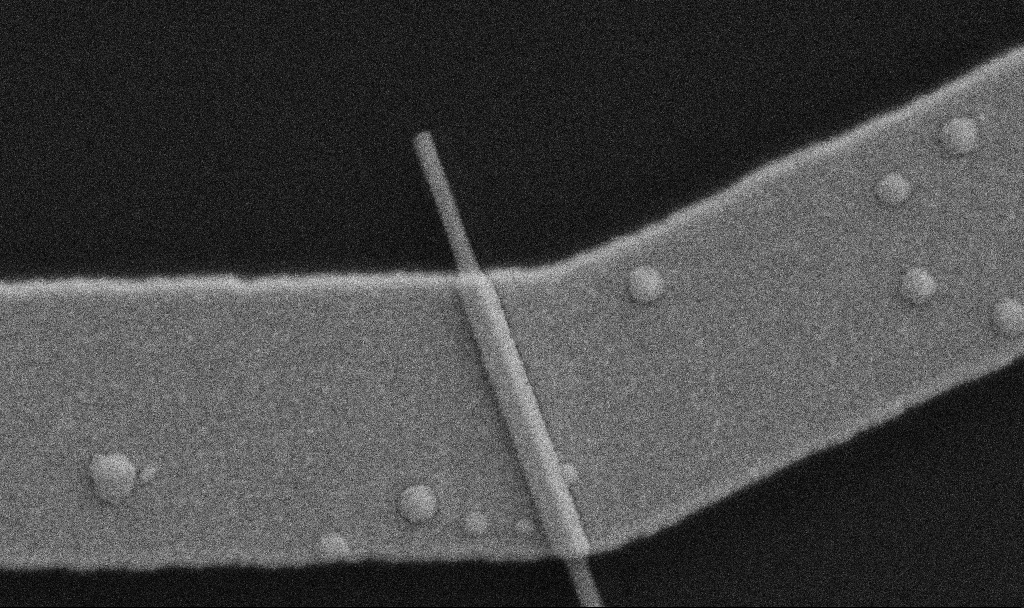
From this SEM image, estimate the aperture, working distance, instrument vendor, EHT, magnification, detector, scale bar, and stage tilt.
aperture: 30 µm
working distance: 10.7 mm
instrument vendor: Zeiss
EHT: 5 kV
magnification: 100 K X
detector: SE2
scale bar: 200 nm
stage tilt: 0°